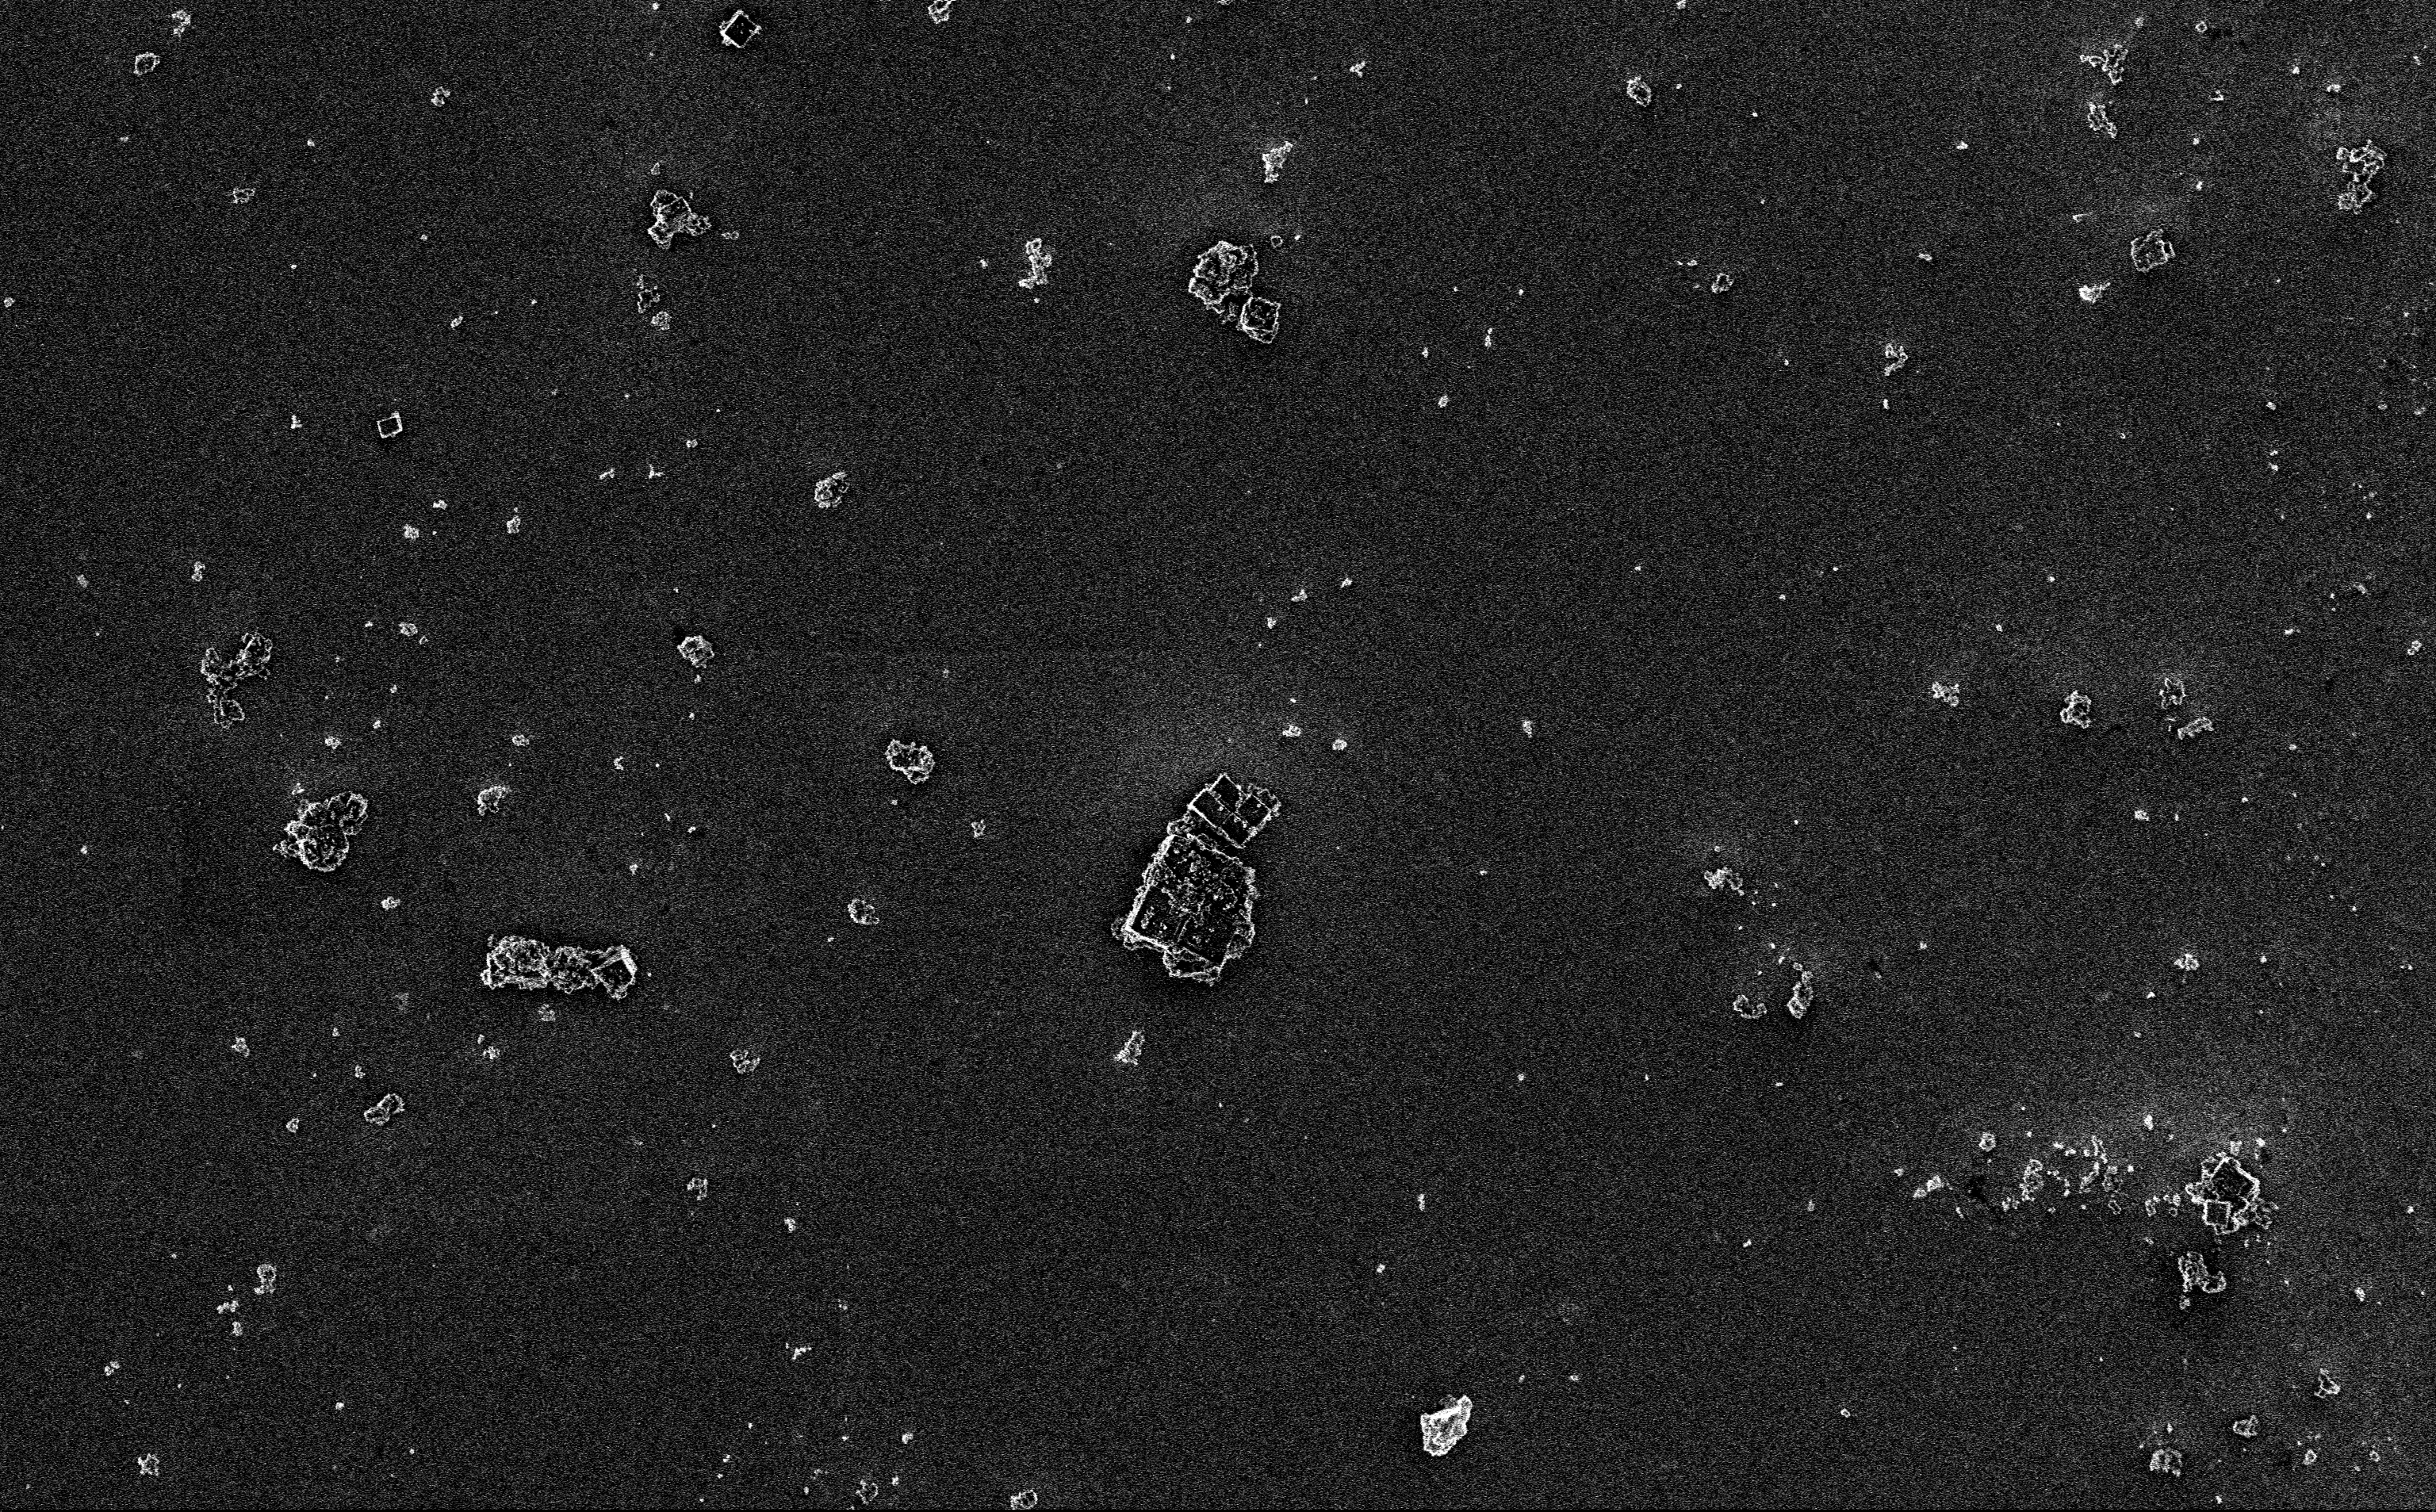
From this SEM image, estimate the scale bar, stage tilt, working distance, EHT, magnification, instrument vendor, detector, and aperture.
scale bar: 10000 nm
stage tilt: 0°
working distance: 3 mm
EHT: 3 kV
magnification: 3.19 K X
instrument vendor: Zeiss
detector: InLens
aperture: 30 µm